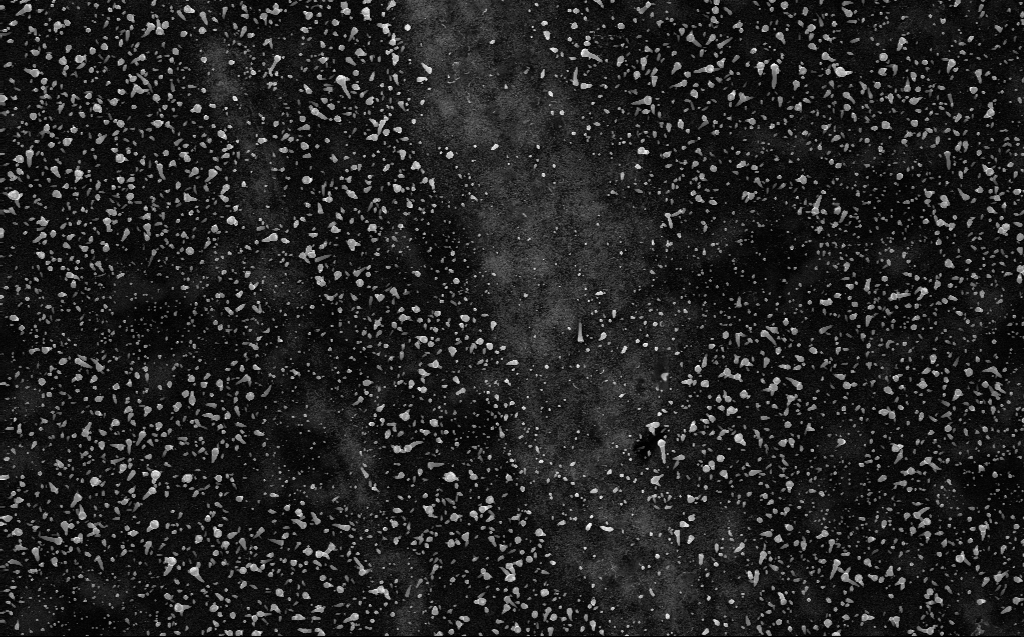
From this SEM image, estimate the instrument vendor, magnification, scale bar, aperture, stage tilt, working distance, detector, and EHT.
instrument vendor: Zeiss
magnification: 13.64 K X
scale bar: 2000 nm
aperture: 30 µm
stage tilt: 0°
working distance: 3 mm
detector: InLens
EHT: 10 kV